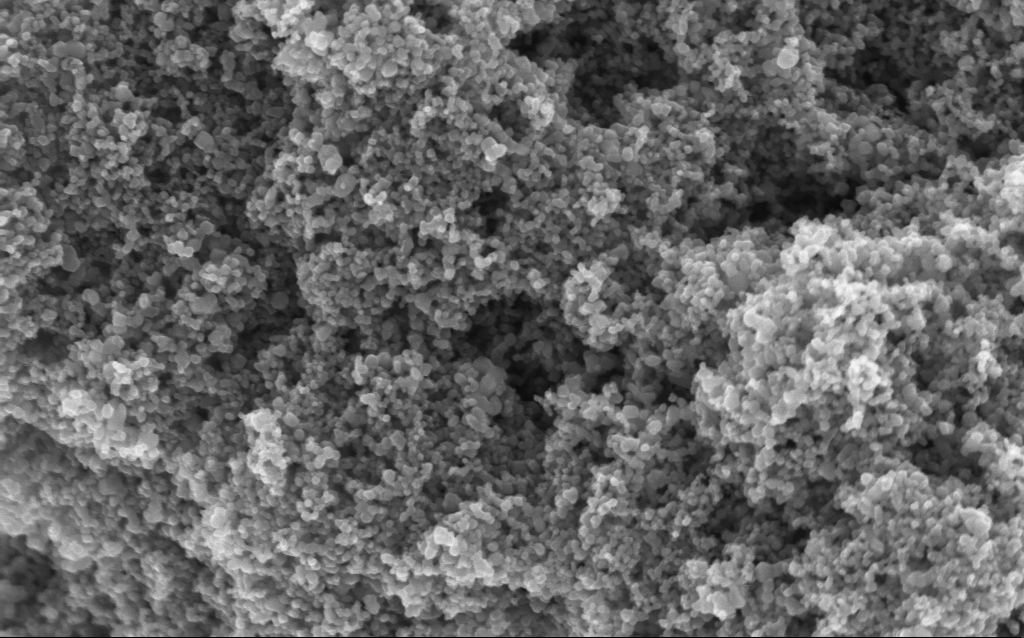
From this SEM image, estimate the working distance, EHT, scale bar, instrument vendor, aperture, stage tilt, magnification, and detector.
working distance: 4.2 mm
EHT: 5 kV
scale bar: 200 nm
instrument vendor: Zeiss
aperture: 30 µm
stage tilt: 0°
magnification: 114.56 K X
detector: InLens